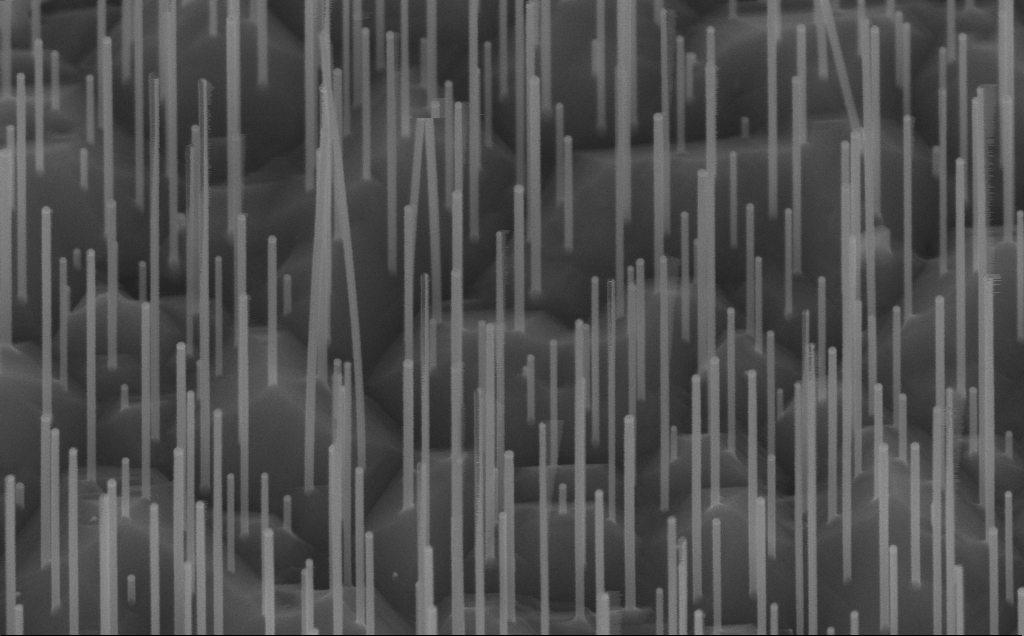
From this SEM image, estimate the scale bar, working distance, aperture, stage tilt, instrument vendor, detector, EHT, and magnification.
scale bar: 200 nm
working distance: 8 mm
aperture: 30 µm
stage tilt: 45°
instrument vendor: Zeiss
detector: InLens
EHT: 10 kV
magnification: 102.84 K X